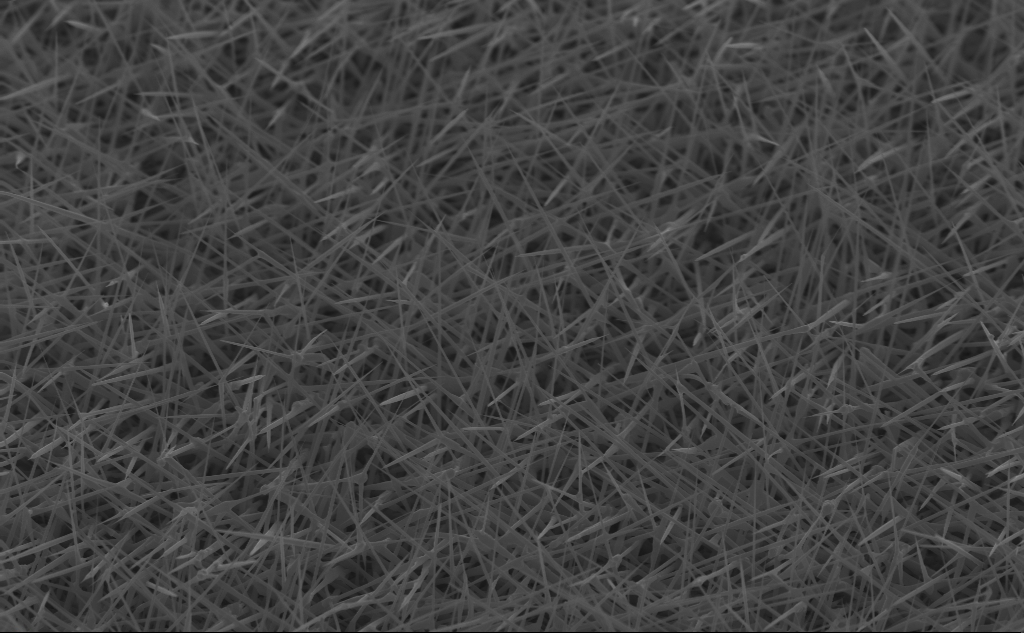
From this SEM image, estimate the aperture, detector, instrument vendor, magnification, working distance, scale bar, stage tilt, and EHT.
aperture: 30 µm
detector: InLens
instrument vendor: Zeiss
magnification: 20 K X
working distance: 5 mm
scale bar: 2000 nm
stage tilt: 45°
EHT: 10 kV